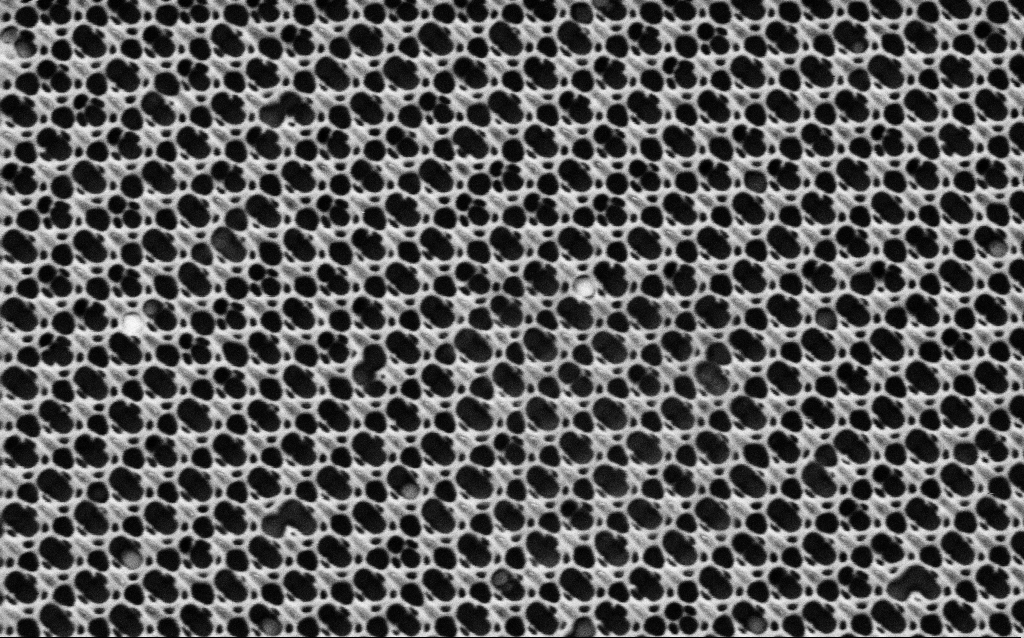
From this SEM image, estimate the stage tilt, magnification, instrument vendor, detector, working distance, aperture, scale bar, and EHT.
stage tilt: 0°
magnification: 38.56 K X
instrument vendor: Zeiss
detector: SE2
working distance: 8.1 mm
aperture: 30 µm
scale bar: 1000 nm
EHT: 1.5 kV